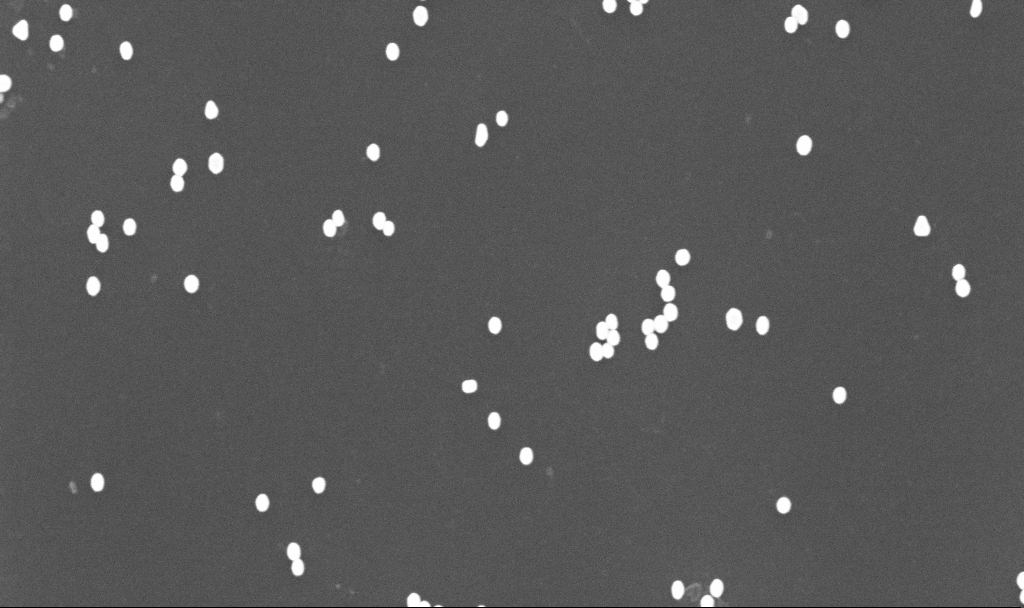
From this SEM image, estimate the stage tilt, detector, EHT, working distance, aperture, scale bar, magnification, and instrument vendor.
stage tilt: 0°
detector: InLens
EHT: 10 kV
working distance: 3.4 mm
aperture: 30 µm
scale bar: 1000 nm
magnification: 70 K X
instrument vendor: Zeiss